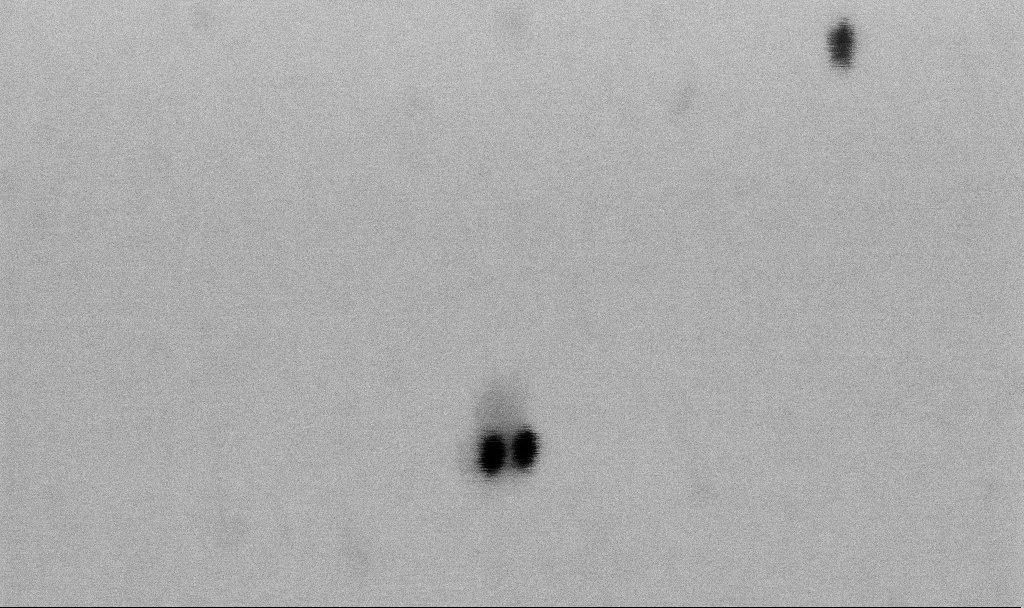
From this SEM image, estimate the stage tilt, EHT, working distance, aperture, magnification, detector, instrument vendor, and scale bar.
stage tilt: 0°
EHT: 2 kV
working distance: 6.6 mm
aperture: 30 µm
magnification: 400 K X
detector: SE2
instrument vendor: Zeiss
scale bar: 100 nm